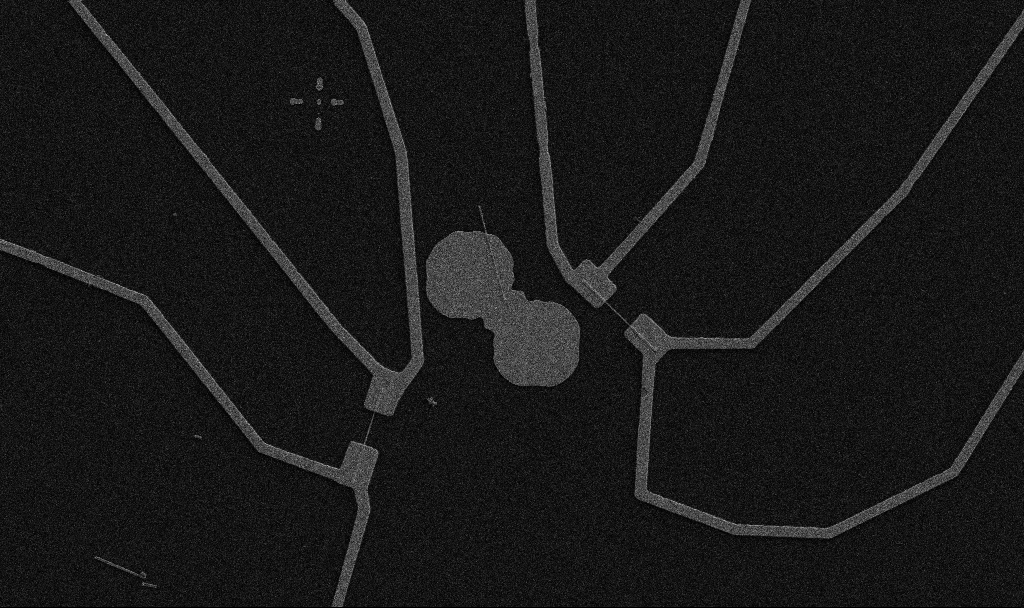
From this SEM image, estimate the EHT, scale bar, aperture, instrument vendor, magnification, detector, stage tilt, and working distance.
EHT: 5 kV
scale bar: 10000 nm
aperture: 30 µm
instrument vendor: Zeiss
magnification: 5 K X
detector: SE2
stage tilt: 0°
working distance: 10.7 mm